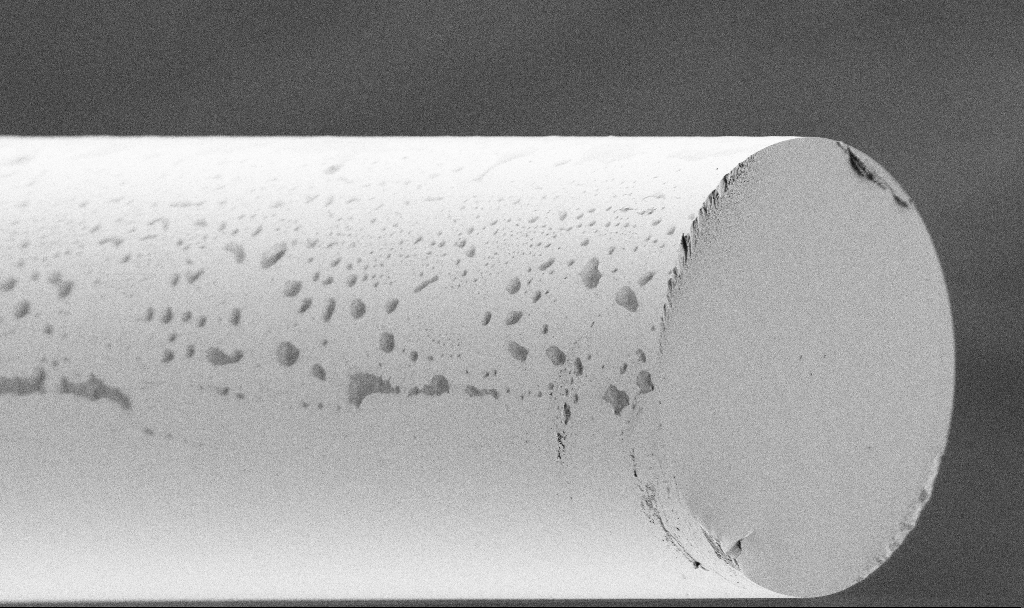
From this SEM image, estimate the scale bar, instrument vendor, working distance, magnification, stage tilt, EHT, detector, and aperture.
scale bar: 20000 nm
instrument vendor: Zeiss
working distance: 3.3 mm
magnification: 1.34 K X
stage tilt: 45°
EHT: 1.5 kV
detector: SE2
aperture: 30 µm